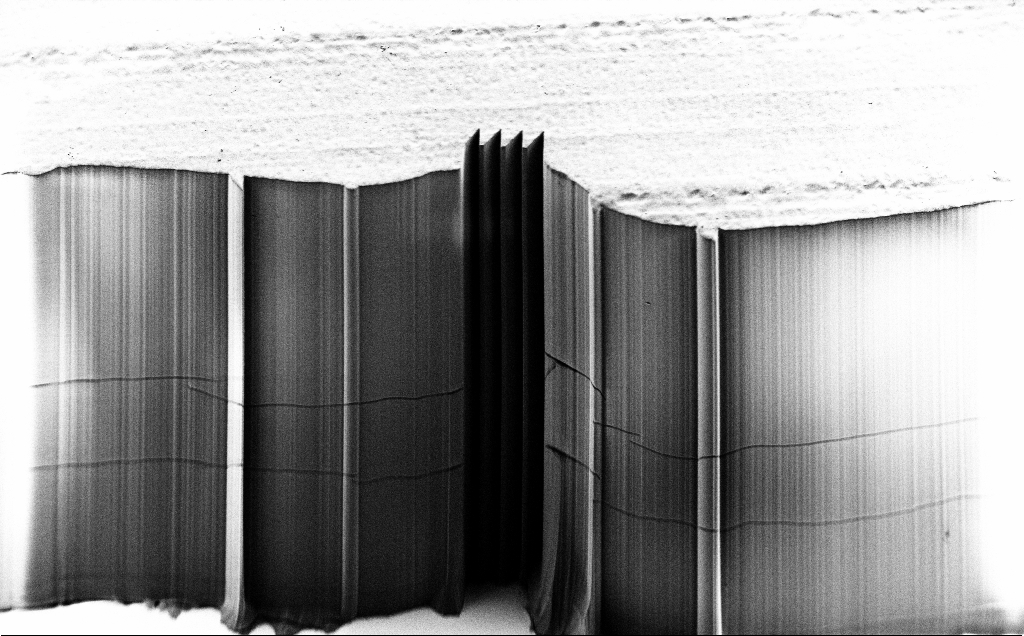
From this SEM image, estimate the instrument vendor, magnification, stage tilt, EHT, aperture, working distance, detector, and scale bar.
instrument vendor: Zeiss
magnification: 0.692 K X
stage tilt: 45°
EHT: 1.2 kV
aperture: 30 µm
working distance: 7 mm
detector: SE2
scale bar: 100000 nm